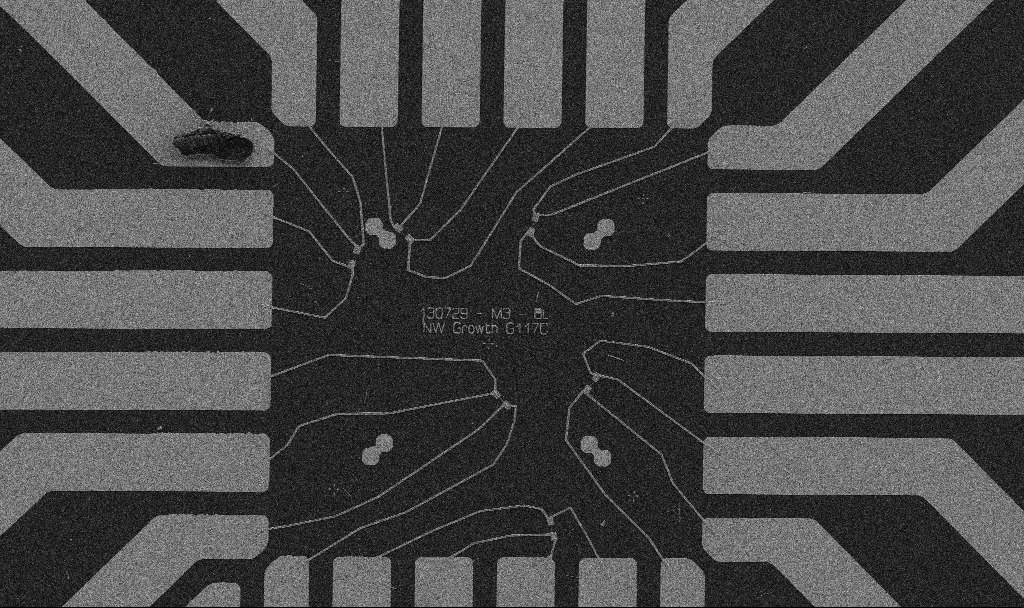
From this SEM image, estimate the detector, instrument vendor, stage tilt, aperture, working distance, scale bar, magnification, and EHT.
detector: SE2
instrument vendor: Zeiss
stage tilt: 0°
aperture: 30 µm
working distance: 10.7 mm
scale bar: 20000 nm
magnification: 1 K X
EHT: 5 kV